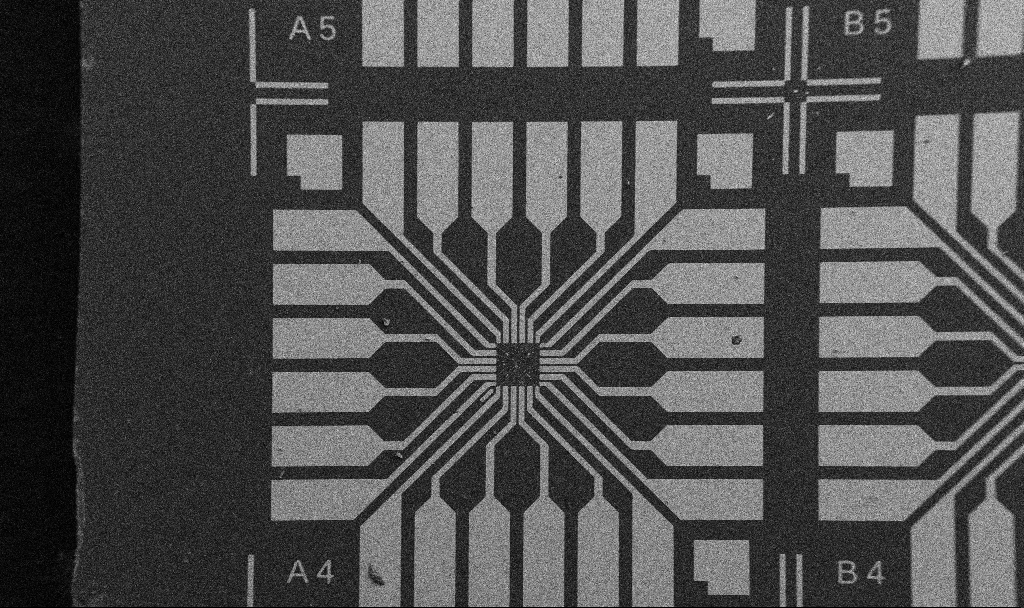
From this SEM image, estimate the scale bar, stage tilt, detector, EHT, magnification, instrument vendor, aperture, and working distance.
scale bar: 200000 nm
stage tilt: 0°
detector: SE2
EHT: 5 kV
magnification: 0.1 K X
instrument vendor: Zeiss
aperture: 30 µm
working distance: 10.7 mm